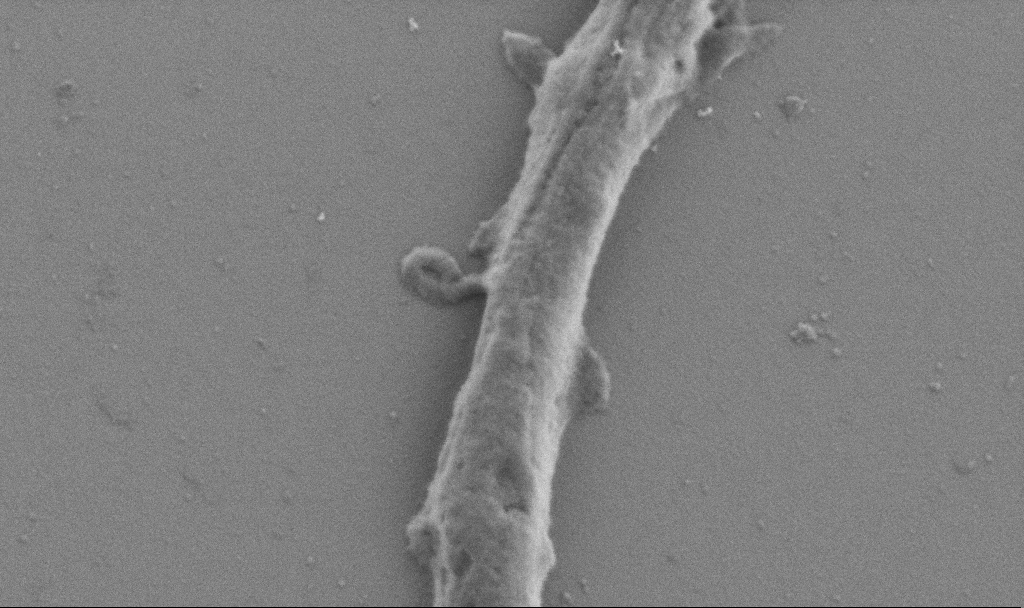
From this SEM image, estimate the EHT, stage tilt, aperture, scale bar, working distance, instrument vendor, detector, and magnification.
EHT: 0.9 kV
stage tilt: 0°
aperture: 30 µm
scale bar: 1000 nm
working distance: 6.9 mm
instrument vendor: Zeiss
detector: SE2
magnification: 50 K X